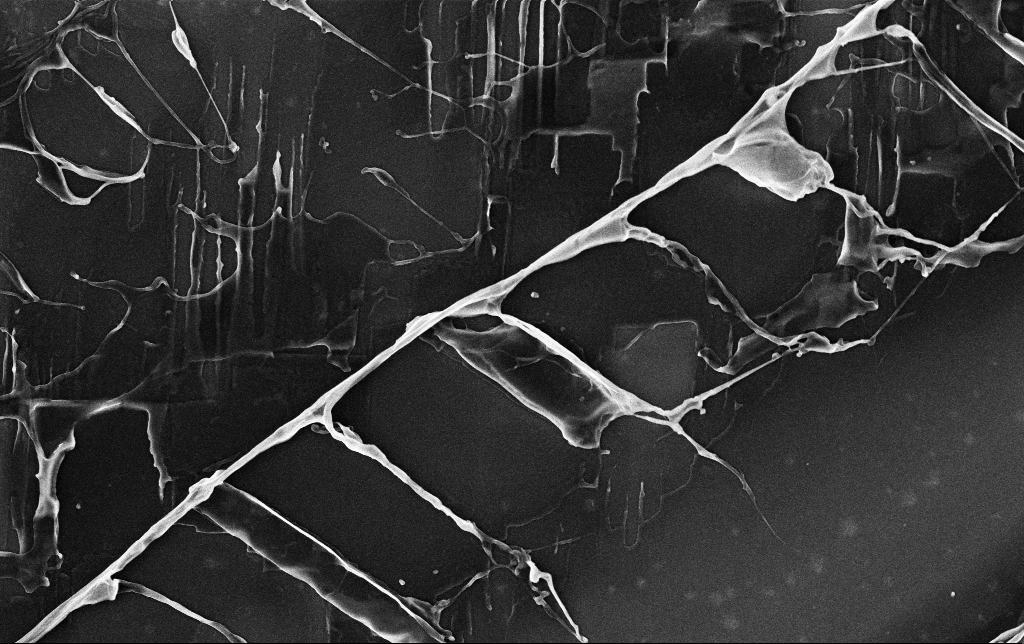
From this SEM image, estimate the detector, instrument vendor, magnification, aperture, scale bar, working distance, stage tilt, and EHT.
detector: InLens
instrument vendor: Zeiss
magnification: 11.88 K X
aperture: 30 µm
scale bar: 2000 nm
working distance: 3.5 mm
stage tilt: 0°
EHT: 3 kV